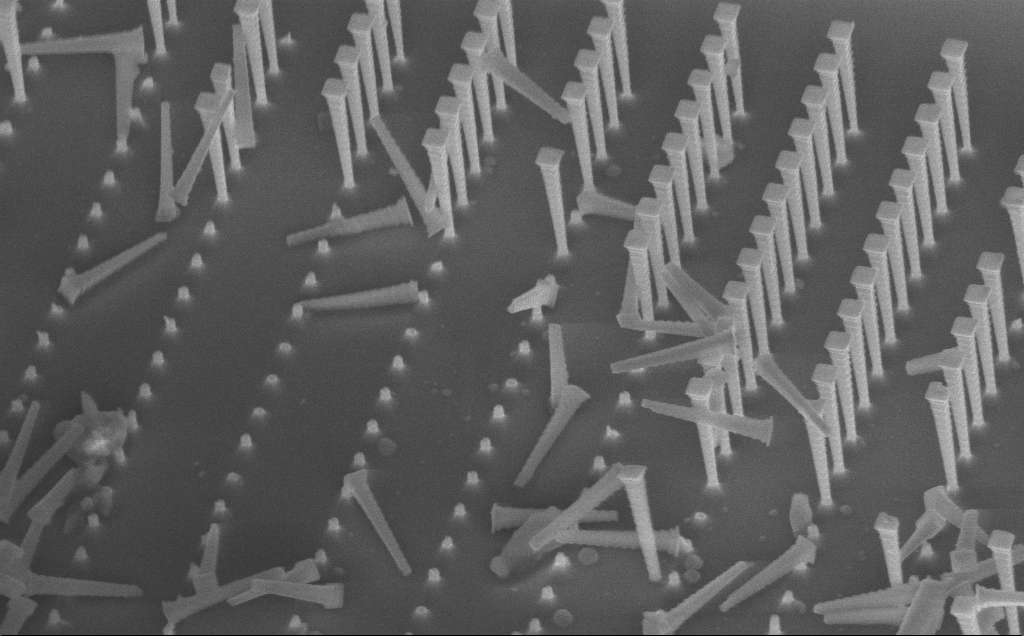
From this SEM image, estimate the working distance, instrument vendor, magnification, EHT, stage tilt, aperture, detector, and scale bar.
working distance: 8 mm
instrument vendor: Zeiss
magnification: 8.73 K X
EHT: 10 kV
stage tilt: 45°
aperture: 30 µm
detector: InLens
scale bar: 2000 nm